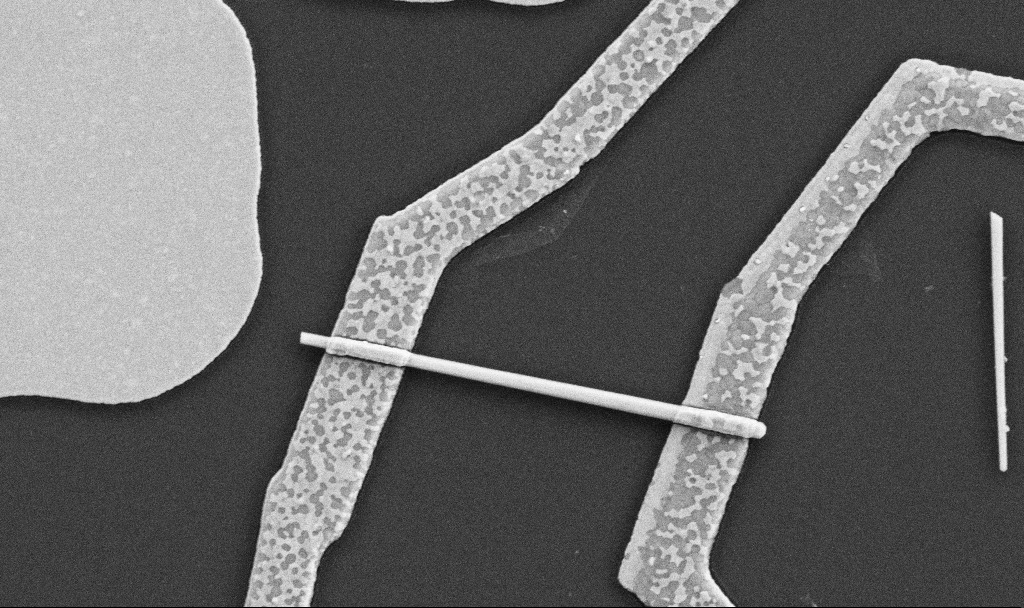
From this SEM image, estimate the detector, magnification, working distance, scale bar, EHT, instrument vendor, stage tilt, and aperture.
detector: SE2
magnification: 30 K X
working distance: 8.7 mm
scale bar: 1000 nm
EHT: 5 kV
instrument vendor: Zeiss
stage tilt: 0°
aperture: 30 µm